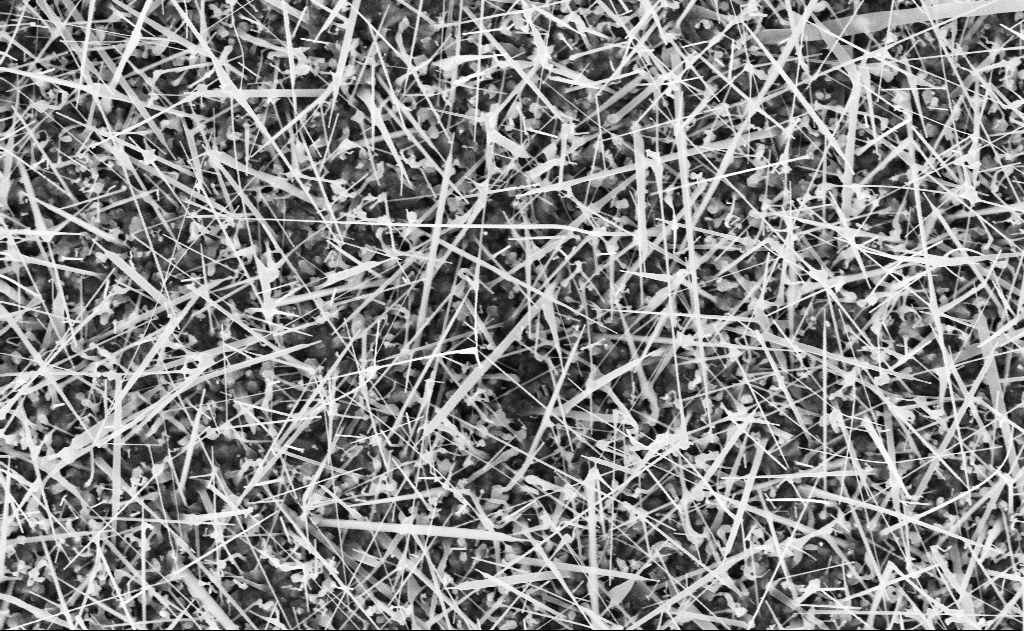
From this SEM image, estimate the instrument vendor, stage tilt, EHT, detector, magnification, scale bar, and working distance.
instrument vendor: Zeiss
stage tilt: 0°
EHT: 10 kV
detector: InLens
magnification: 20 K X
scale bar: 1000 nm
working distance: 20 mm